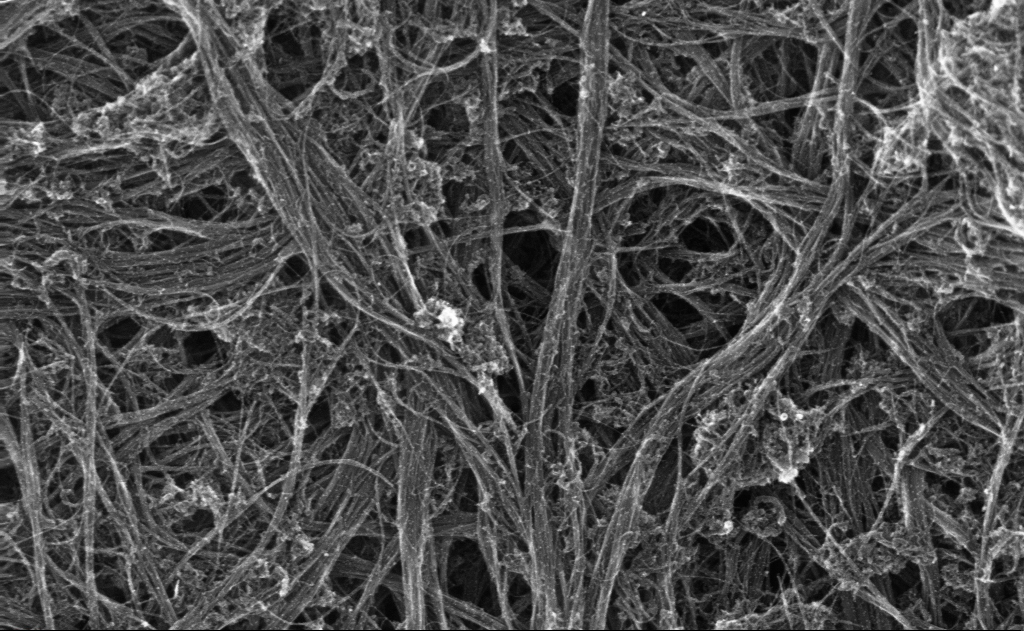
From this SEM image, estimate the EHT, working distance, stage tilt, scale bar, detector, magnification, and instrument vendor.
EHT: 10 kV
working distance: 3 mm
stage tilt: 0°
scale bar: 200 nm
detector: InLens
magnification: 135.76 K X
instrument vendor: Zeiss